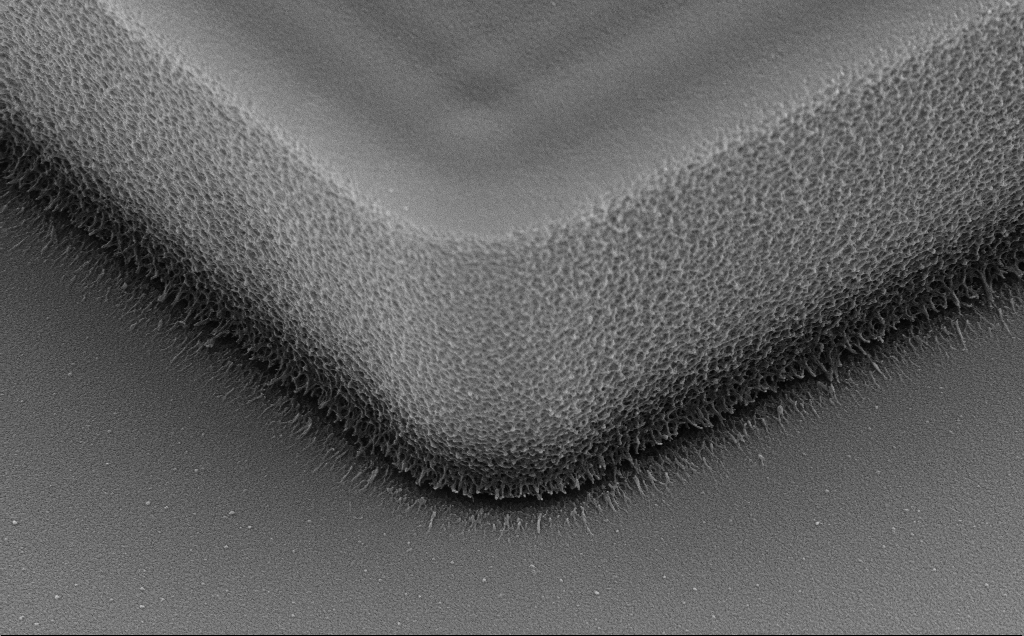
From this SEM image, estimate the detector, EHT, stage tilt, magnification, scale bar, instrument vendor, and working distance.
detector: SE2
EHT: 10 kV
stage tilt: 35.3°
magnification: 21.99 K X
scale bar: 2000 nm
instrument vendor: Zeiss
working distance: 11 mm